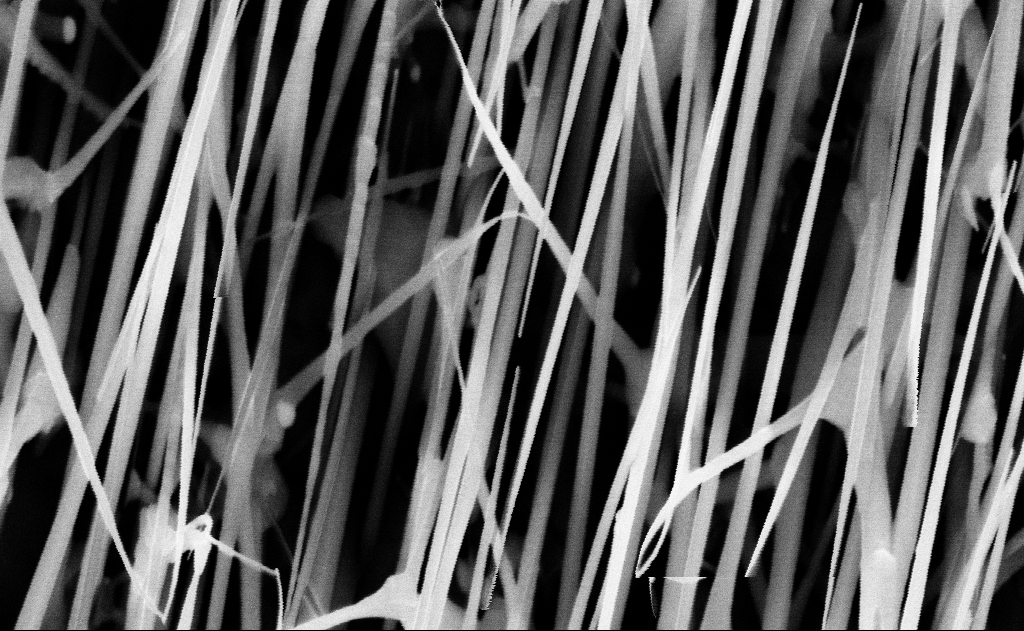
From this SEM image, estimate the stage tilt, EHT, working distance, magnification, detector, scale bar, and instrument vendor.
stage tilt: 0°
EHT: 10 kV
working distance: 16 mm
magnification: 80 K X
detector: InLens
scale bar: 200 nm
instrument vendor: Zeiss